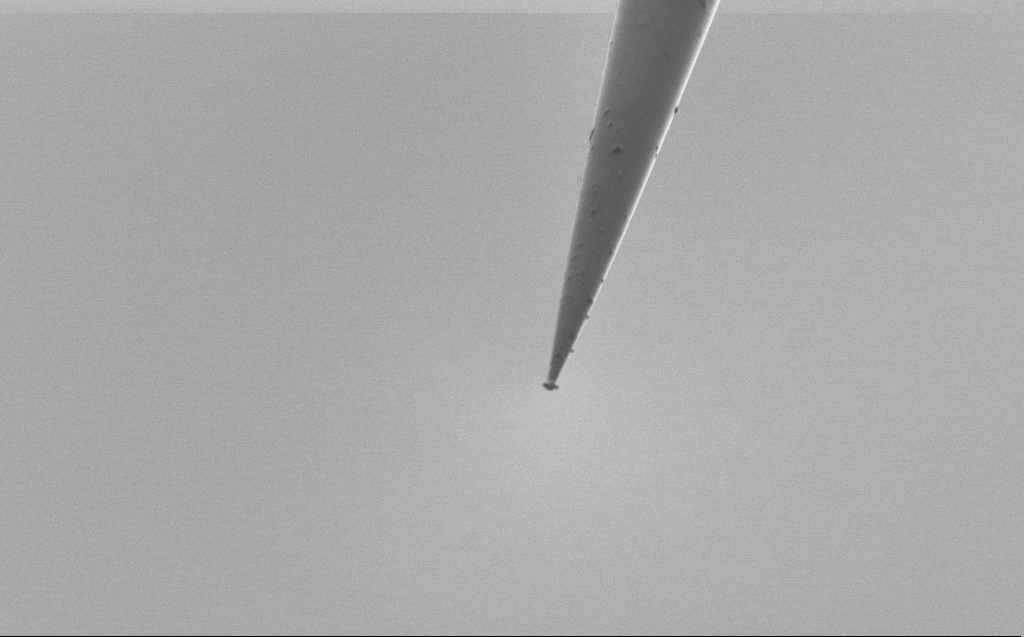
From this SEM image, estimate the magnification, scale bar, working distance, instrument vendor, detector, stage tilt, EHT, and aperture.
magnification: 10 K X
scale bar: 2000 nm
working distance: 5 mm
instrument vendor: Zeiss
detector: SE2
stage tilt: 45°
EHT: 1 kV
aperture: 30 µm